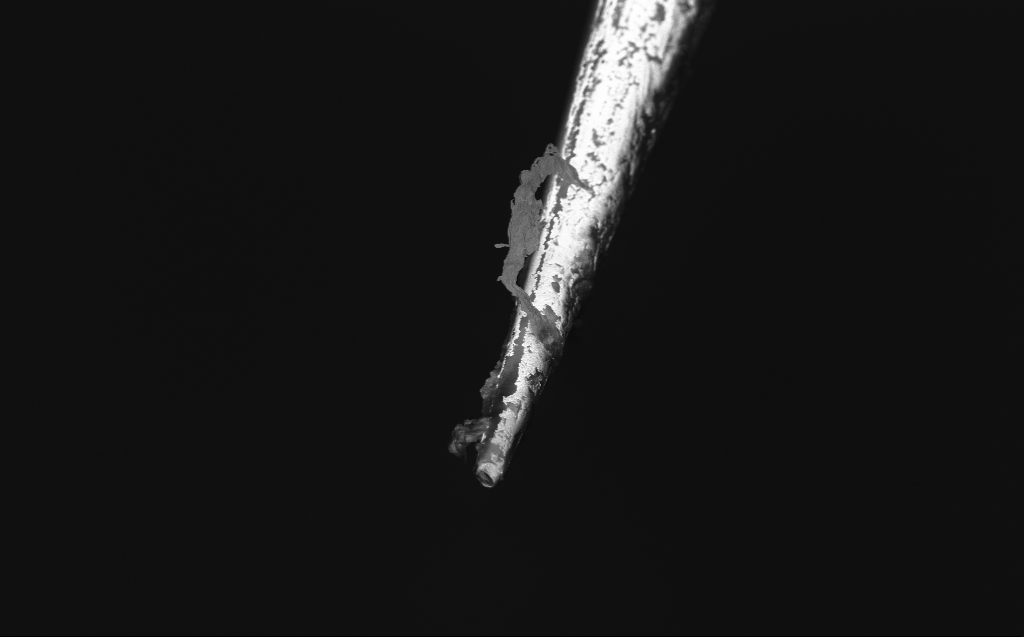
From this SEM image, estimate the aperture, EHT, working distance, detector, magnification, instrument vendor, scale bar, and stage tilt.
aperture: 30 µm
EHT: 1 kV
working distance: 4 mm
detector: InLens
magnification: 3.34 K X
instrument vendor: Zeiss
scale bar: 10000 nm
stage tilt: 45°